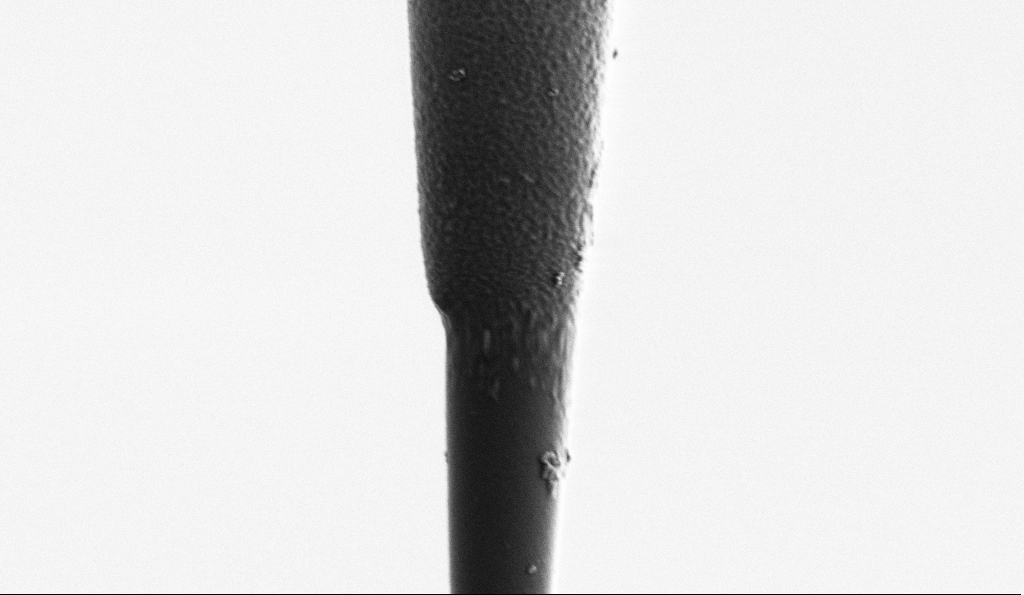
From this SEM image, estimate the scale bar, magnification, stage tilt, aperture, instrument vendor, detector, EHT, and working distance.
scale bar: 2000 nm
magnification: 25 K X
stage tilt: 0°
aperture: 30 µm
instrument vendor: Zeiss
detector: SE2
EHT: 1 kV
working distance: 6.5 mm